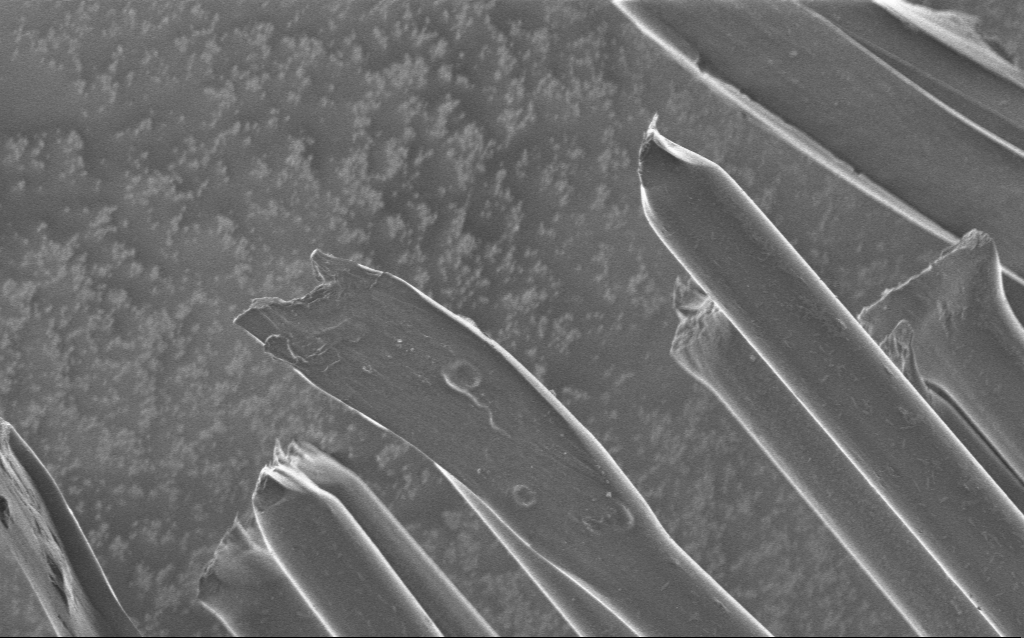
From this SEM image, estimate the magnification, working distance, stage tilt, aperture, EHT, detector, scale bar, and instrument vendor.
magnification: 2.43 K X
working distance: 5 mm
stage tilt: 0°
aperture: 30 µm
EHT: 1 kV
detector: InLens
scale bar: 10000 nm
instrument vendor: Zeiss